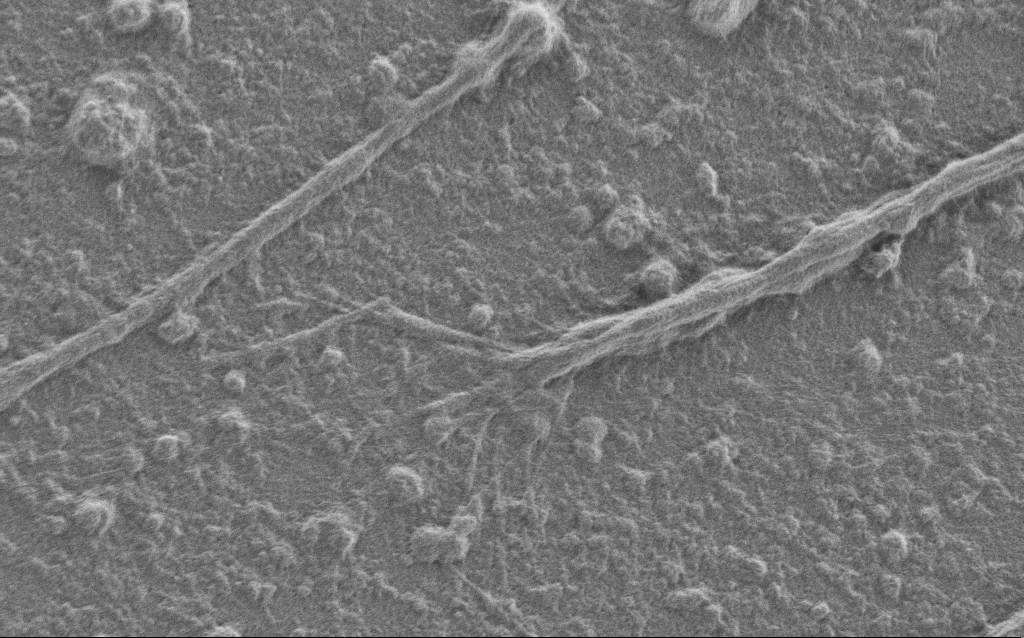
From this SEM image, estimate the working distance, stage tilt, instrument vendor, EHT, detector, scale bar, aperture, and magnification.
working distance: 6 mm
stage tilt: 0°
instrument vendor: Zeiss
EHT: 1 kV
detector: SE2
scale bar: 2000 nm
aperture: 30 µm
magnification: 7.5 K X